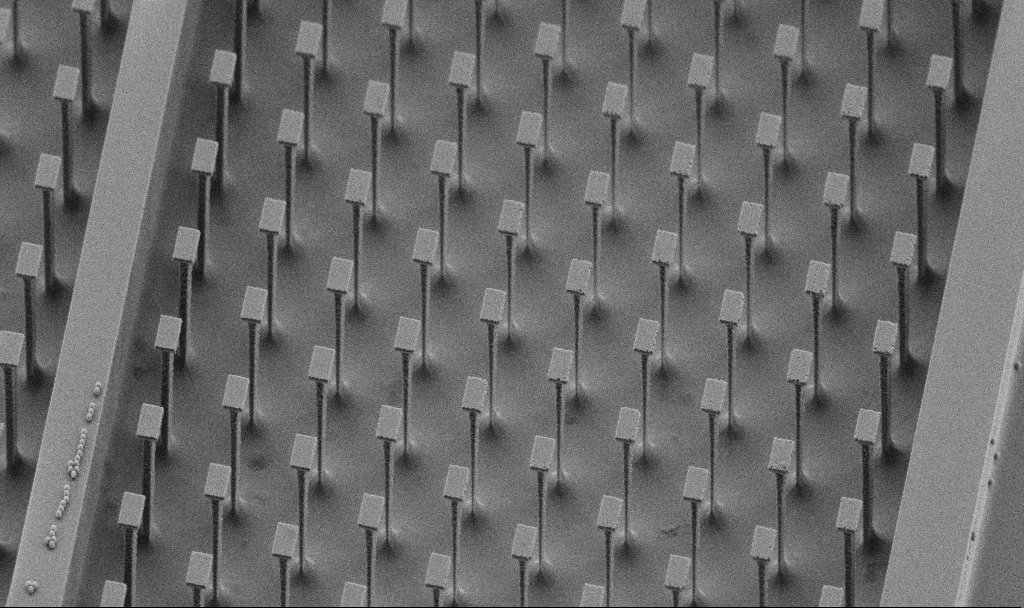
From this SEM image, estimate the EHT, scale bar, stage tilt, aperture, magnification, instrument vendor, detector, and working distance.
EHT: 5 kV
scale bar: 10000 nm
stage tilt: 45°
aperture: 30 µm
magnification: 2.76 K X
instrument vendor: Zeiss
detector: SE2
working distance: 5.9 mm